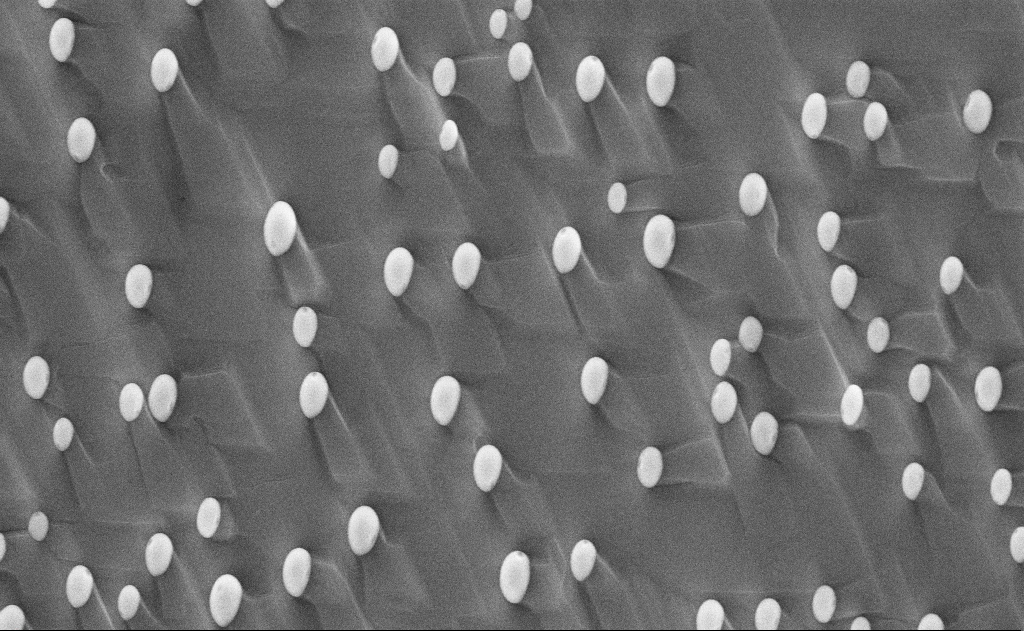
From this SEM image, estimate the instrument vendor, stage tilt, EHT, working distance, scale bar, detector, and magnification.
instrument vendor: Zeiss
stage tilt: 0°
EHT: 10 kV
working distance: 12 mm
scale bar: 2000 nm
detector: InLens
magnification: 20 K X